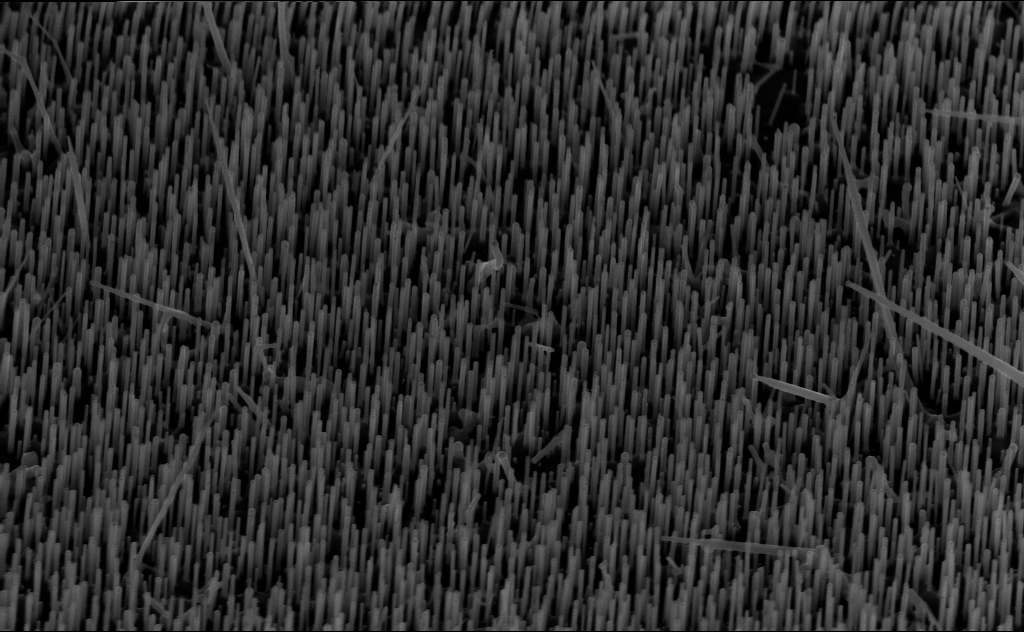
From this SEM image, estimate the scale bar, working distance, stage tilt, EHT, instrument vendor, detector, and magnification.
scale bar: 1000 nm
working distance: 6 mm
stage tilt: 45°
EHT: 10 kV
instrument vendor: Zeiss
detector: InLens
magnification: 40 K X